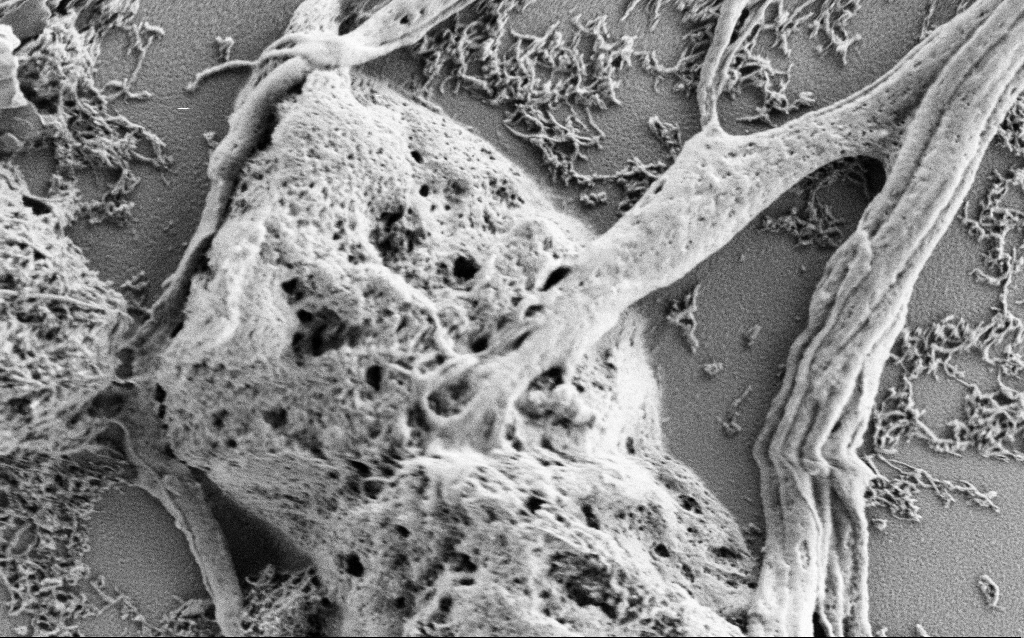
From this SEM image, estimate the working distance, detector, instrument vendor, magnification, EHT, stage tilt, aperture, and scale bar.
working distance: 4 mm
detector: SE2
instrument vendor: Zeiss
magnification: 25 K X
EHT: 1 kV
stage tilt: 0°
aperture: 30 µm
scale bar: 1000 nm